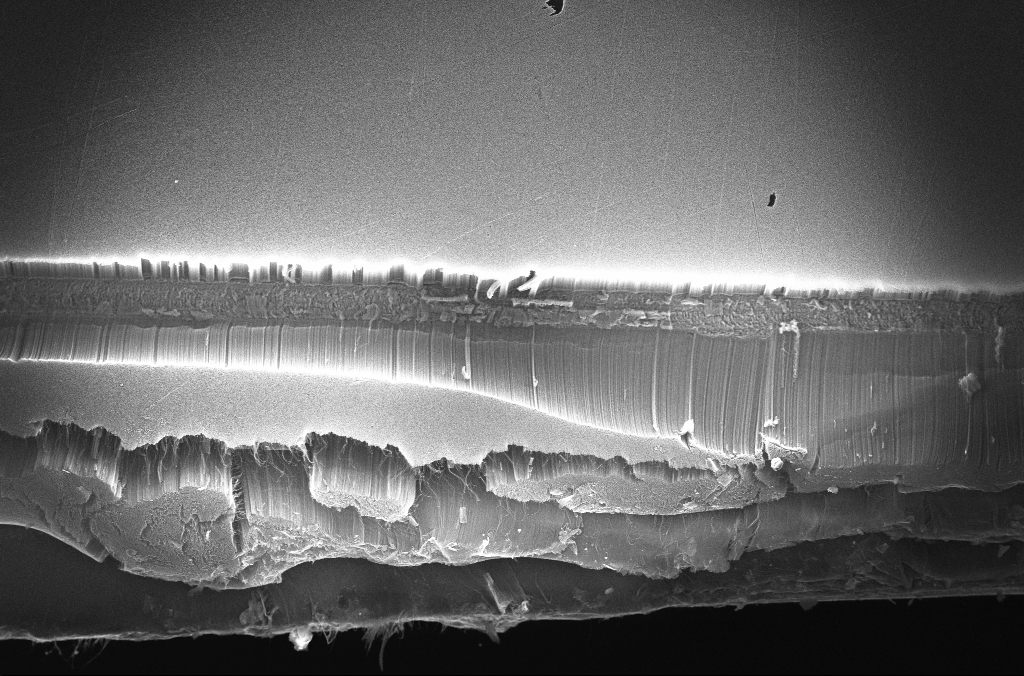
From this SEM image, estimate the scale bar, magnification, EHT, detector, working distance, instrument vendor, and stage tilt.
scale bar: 100000 nm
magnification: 0.2 K X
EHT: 20 kV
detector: InLens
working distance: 4 mm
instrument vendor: Zeiss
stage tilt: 0°